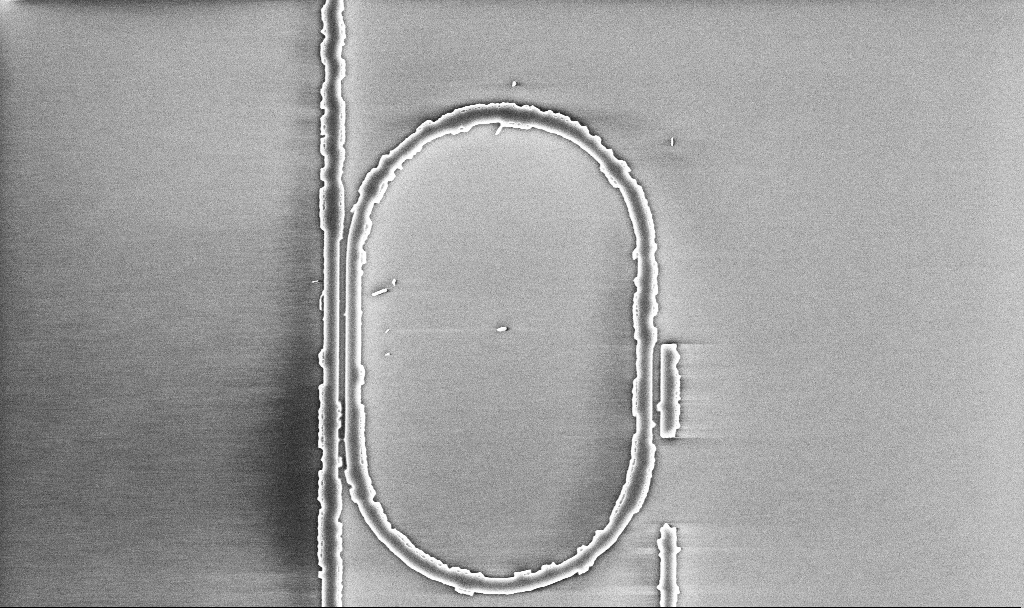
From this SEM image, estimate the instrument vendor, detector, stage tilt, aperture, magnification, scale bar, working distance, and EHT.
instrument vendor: Zeiss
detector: InLens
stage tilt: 0°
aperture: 30 µm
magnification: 11.35 K X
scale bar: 2000 nm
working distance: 10.1 mm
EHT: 5 kV